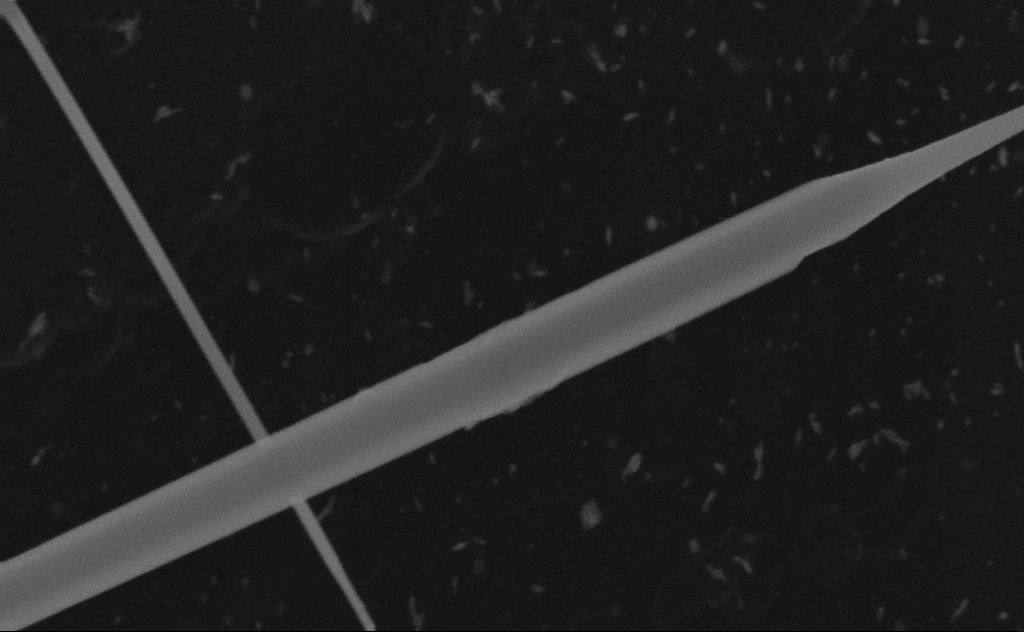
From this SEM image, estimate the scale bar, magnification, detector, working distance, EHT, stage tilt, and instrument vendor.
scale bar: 200 nm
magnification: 177.11 K X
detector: SE2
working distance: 8 mm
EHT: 20 kV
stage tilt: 0°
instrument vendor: Zeiss